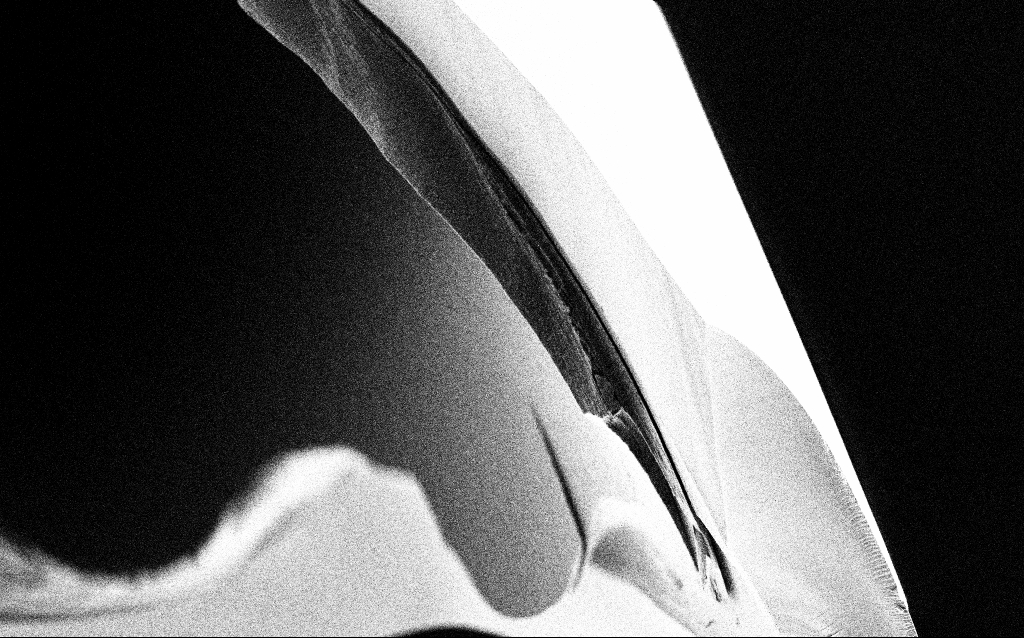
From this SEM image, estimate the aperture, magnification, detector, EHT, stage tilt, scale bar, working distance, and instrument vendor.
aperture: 30 µm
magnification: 10 K X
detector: SE2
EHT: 1 kV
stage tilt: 45°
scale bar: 2000 nm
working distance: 6.4 mm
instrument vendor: Zeiss